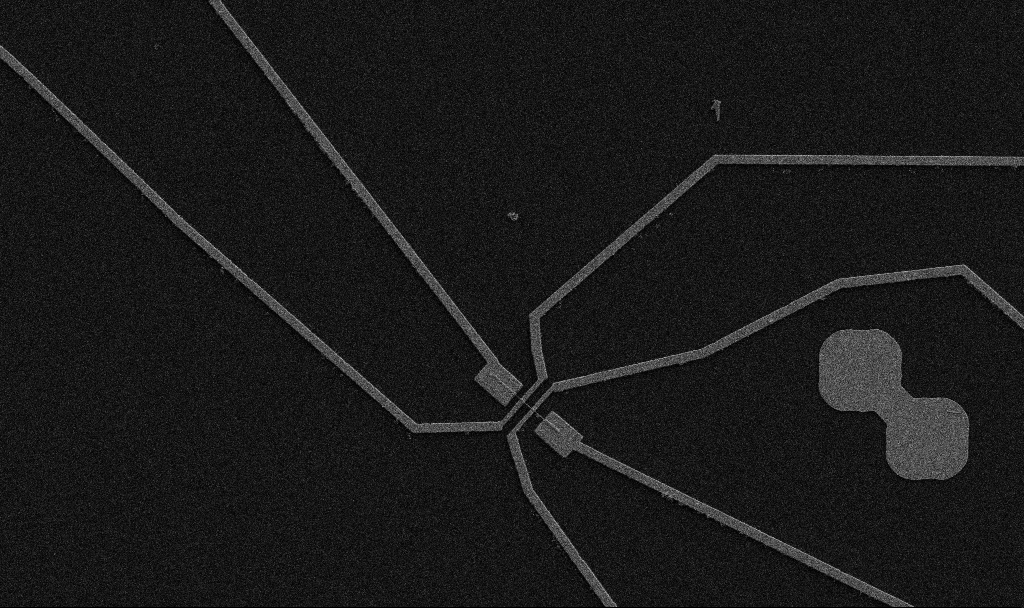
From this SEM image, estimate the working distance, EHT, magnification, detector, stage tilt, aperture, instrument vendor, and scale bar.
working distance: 10.7 mm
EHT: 5 kV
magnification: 5 K X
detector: SE2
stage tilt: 0°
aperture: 30 µm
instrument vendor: Zeiss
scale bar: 10000 nm